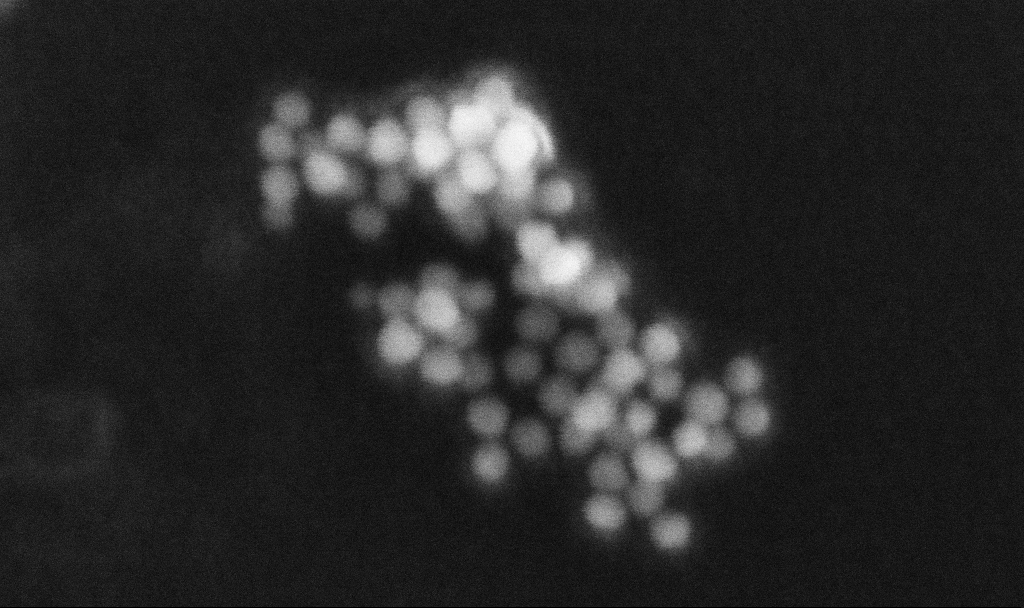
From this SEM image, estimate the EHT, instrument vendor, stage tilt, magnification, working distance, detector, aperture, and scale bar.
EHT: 10 kV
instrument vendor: Zeiss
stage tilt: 0°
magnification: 788.69 K X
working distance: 3.2 mm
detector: InLens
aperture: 30 µm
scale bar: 20 nm